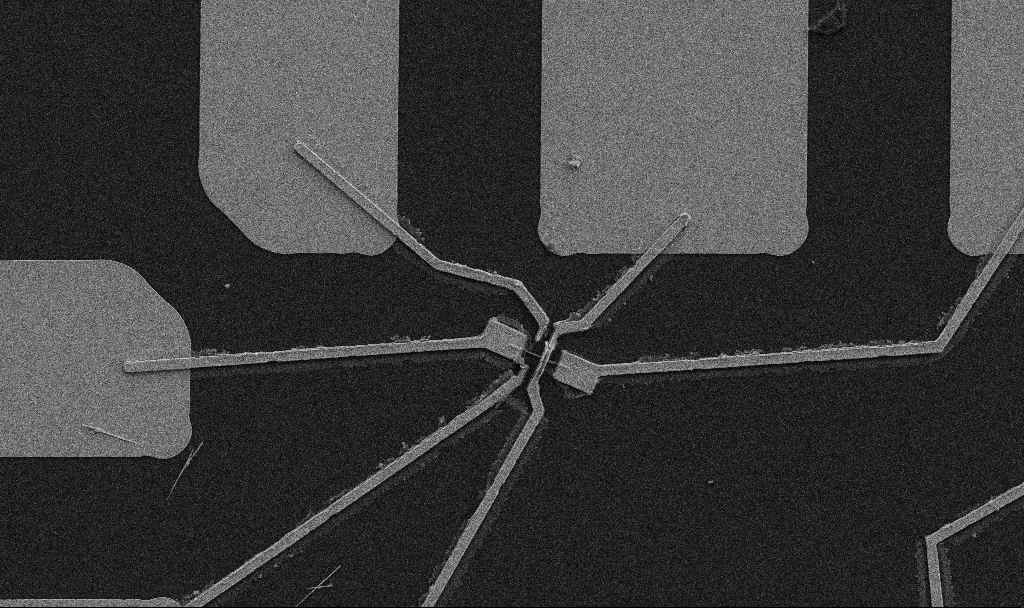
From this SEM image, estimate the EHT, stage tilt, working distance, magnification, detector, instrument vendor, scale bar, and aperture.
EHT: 5 kV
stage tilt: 0°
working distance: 10.7 mm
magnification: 5 K X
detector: SE2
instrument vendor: Zeiss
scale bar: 10000 nm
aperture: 30 µm